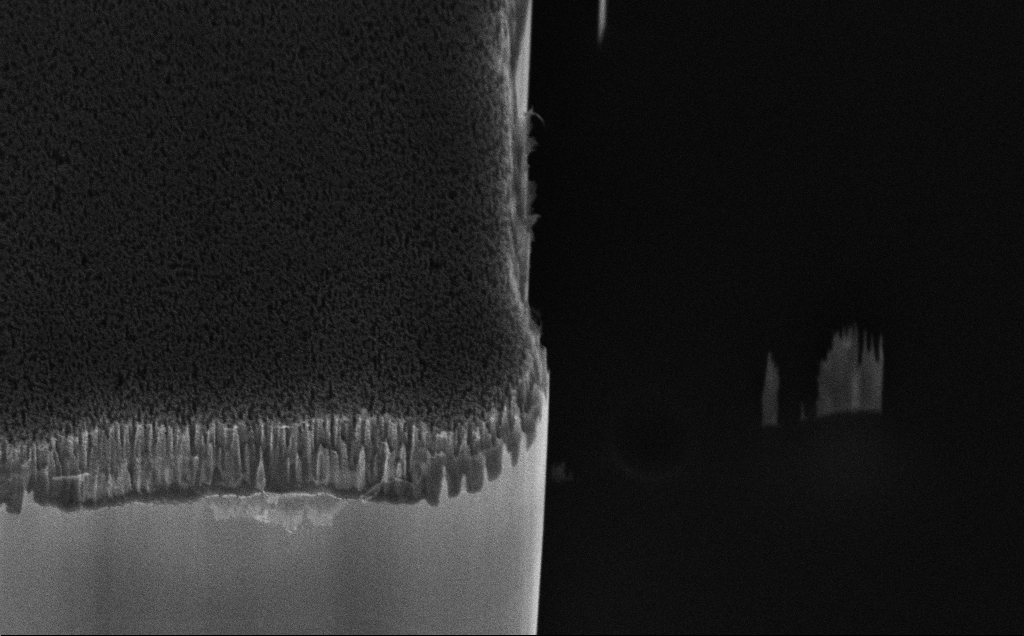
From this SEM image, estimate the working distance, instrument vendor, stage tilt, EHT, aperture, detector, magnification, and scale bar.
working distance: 11 mm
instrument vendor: Zeiss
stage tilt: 45°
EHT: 10 kV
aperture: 30 µm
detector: InLens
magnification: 28.32 K X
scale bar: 1000 nm